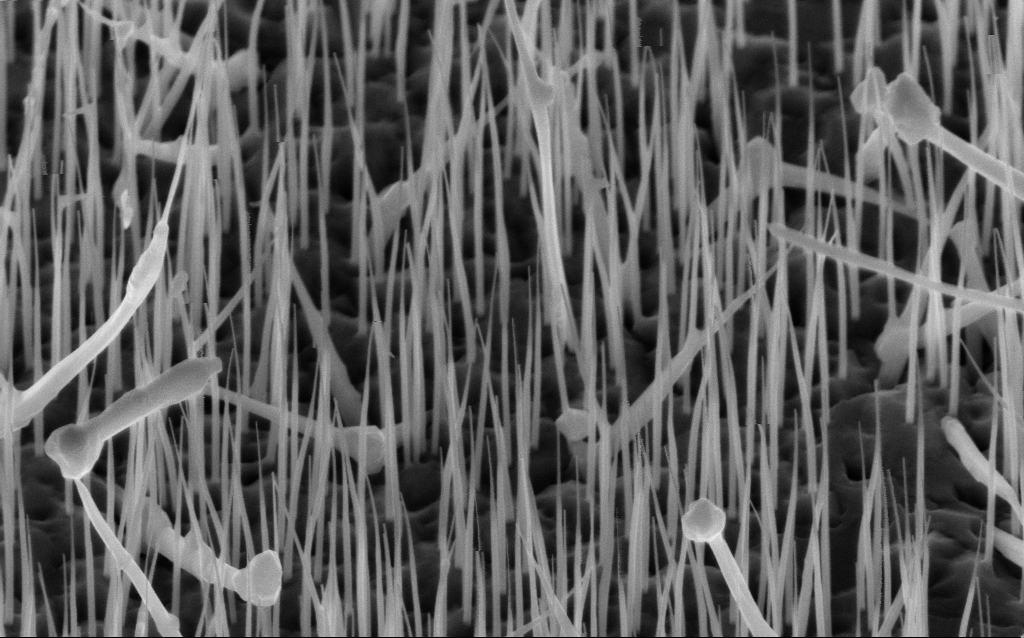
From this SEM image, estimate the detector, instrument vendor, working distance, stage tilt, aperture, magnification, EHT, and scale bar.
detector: InLens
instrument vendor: Zeiss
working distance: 6 mm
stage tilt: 45°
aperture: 30 µm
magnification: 40 K X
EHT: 10 kV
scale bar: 1000 nm